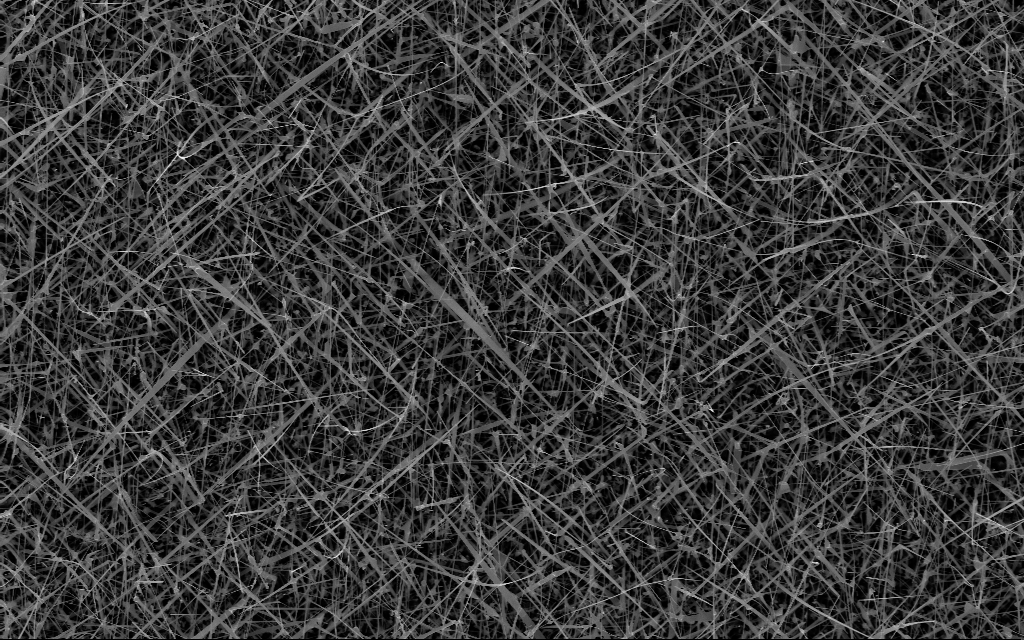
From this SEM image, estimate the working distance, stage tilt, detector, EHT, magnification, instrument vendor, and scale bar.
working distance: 7 mm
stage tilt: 0°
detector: InLens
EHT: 10 kV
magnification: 10 K X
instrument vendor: Zeiss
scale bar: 2000 nm